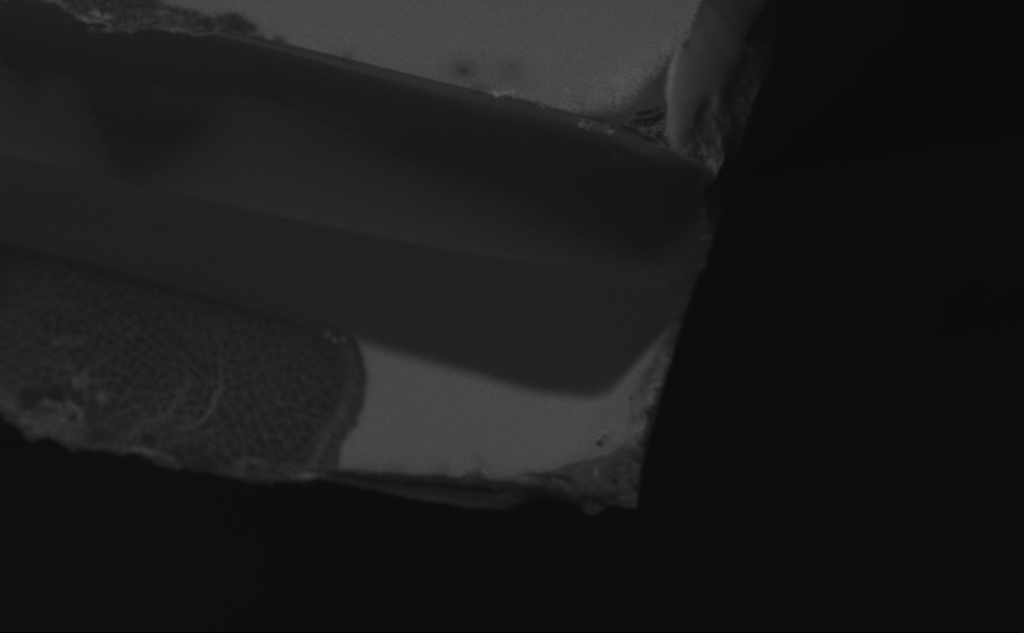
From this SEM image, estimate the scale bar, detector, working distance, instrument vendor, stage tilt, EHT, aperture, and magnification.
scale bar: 100000 nm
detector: InLens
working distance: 5 mm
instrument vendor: Zeiss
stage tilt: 45°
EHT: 10 kV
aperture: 30 µm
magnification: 0.461 K X